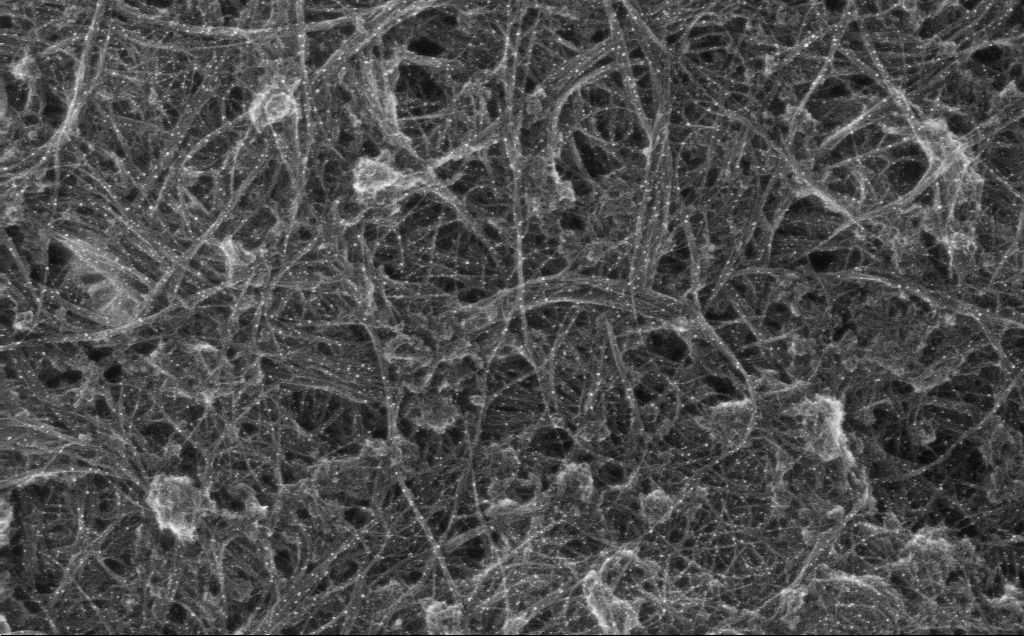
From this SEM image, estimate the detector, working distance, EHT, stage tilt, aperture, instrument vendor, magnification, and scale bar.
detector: InLens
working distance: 3 mm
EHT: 10 kV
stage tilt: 0°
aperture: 30 µm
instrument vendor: Zeiss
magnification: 82.99 K X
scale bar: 200 nm